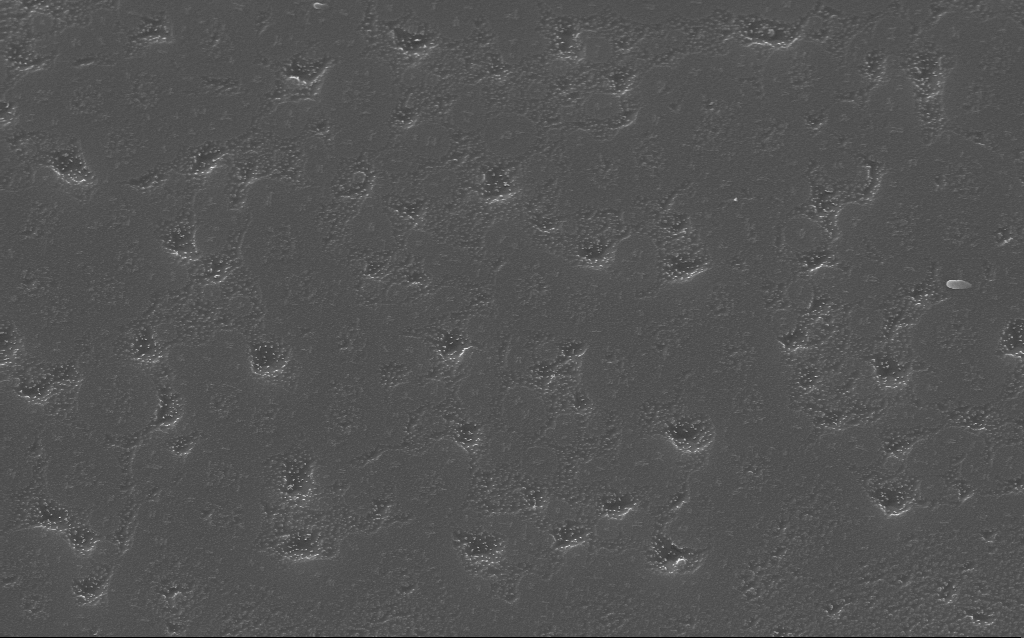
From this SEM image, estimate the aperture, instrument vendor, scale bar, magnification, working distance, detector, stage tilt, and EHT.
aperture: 30 µm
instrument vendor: Zeiss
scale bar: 2000 nm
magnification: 24.23 K X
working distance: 6 mm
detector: InLens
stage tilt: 21.3°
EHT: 10 kV